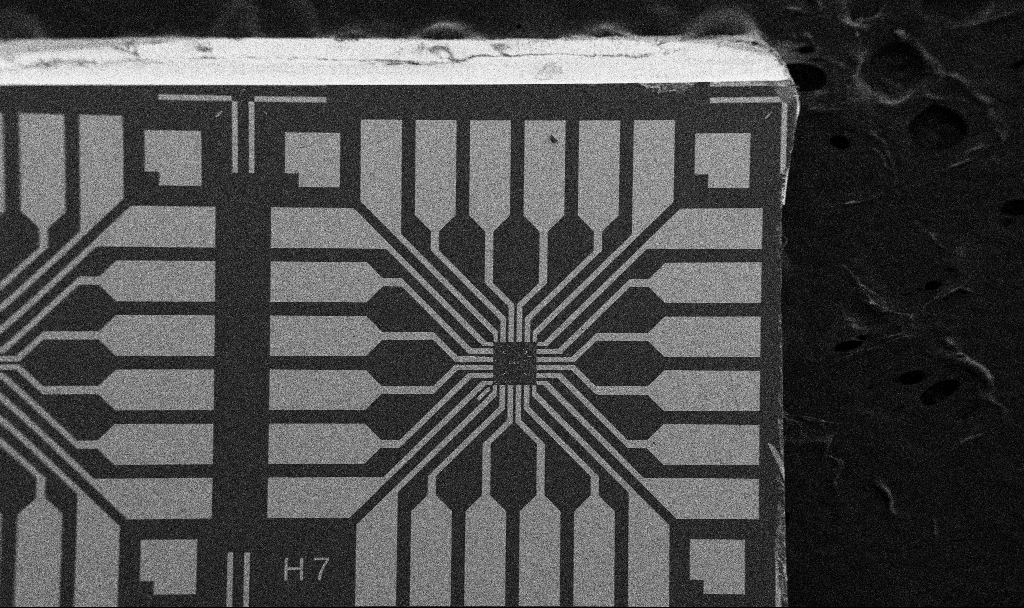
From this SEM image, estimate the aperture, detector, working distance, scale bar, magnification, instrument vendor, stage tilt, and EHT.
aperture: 30 µm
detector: SE2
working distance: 10.7 mm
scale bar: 200000 nm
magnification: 0.1 K X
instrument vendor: Zeiss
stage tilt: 0°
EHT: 5 kV